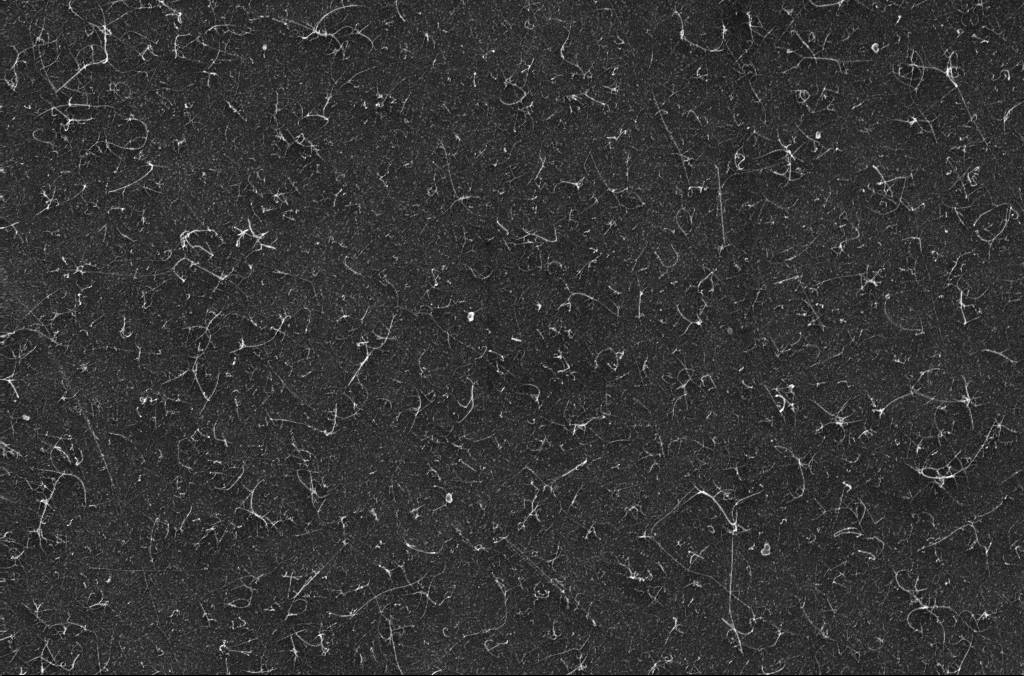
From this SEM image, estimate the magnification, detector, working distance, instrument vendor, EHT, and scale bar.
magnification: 45.51 K X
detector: InLens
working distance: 3.4 mm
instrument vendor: Zeiss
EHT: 10 kV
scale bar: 1000 nm